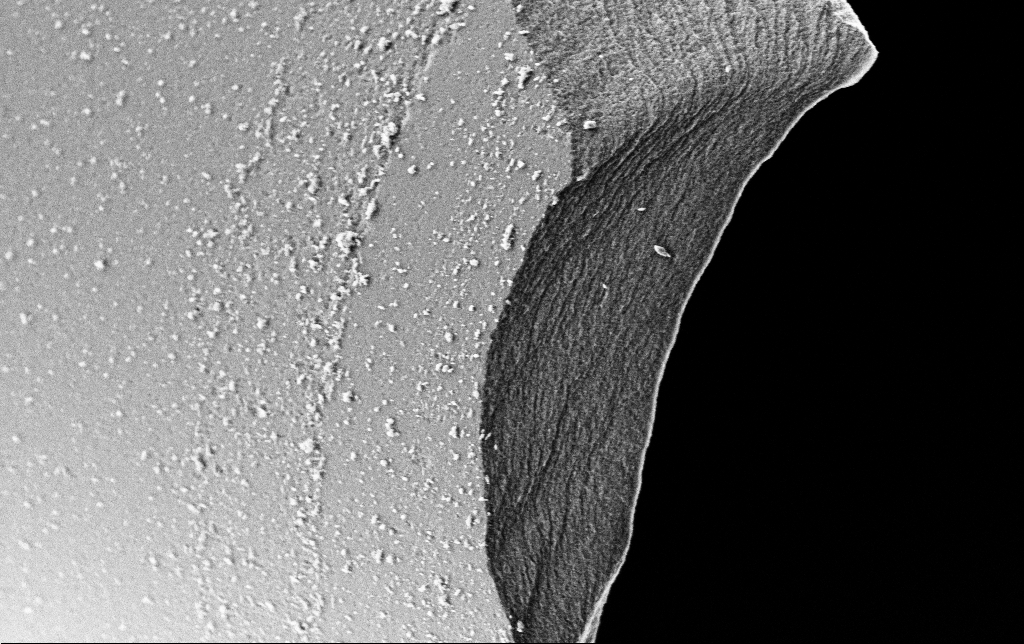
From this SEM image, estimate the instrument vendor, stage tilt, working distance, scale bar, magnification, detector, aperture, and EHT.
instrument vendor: Zeiss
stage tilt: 45°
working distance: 7.1 mm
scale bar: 1000 nm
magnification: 15 K X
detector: SE2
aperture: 30 µm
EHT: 2 kV